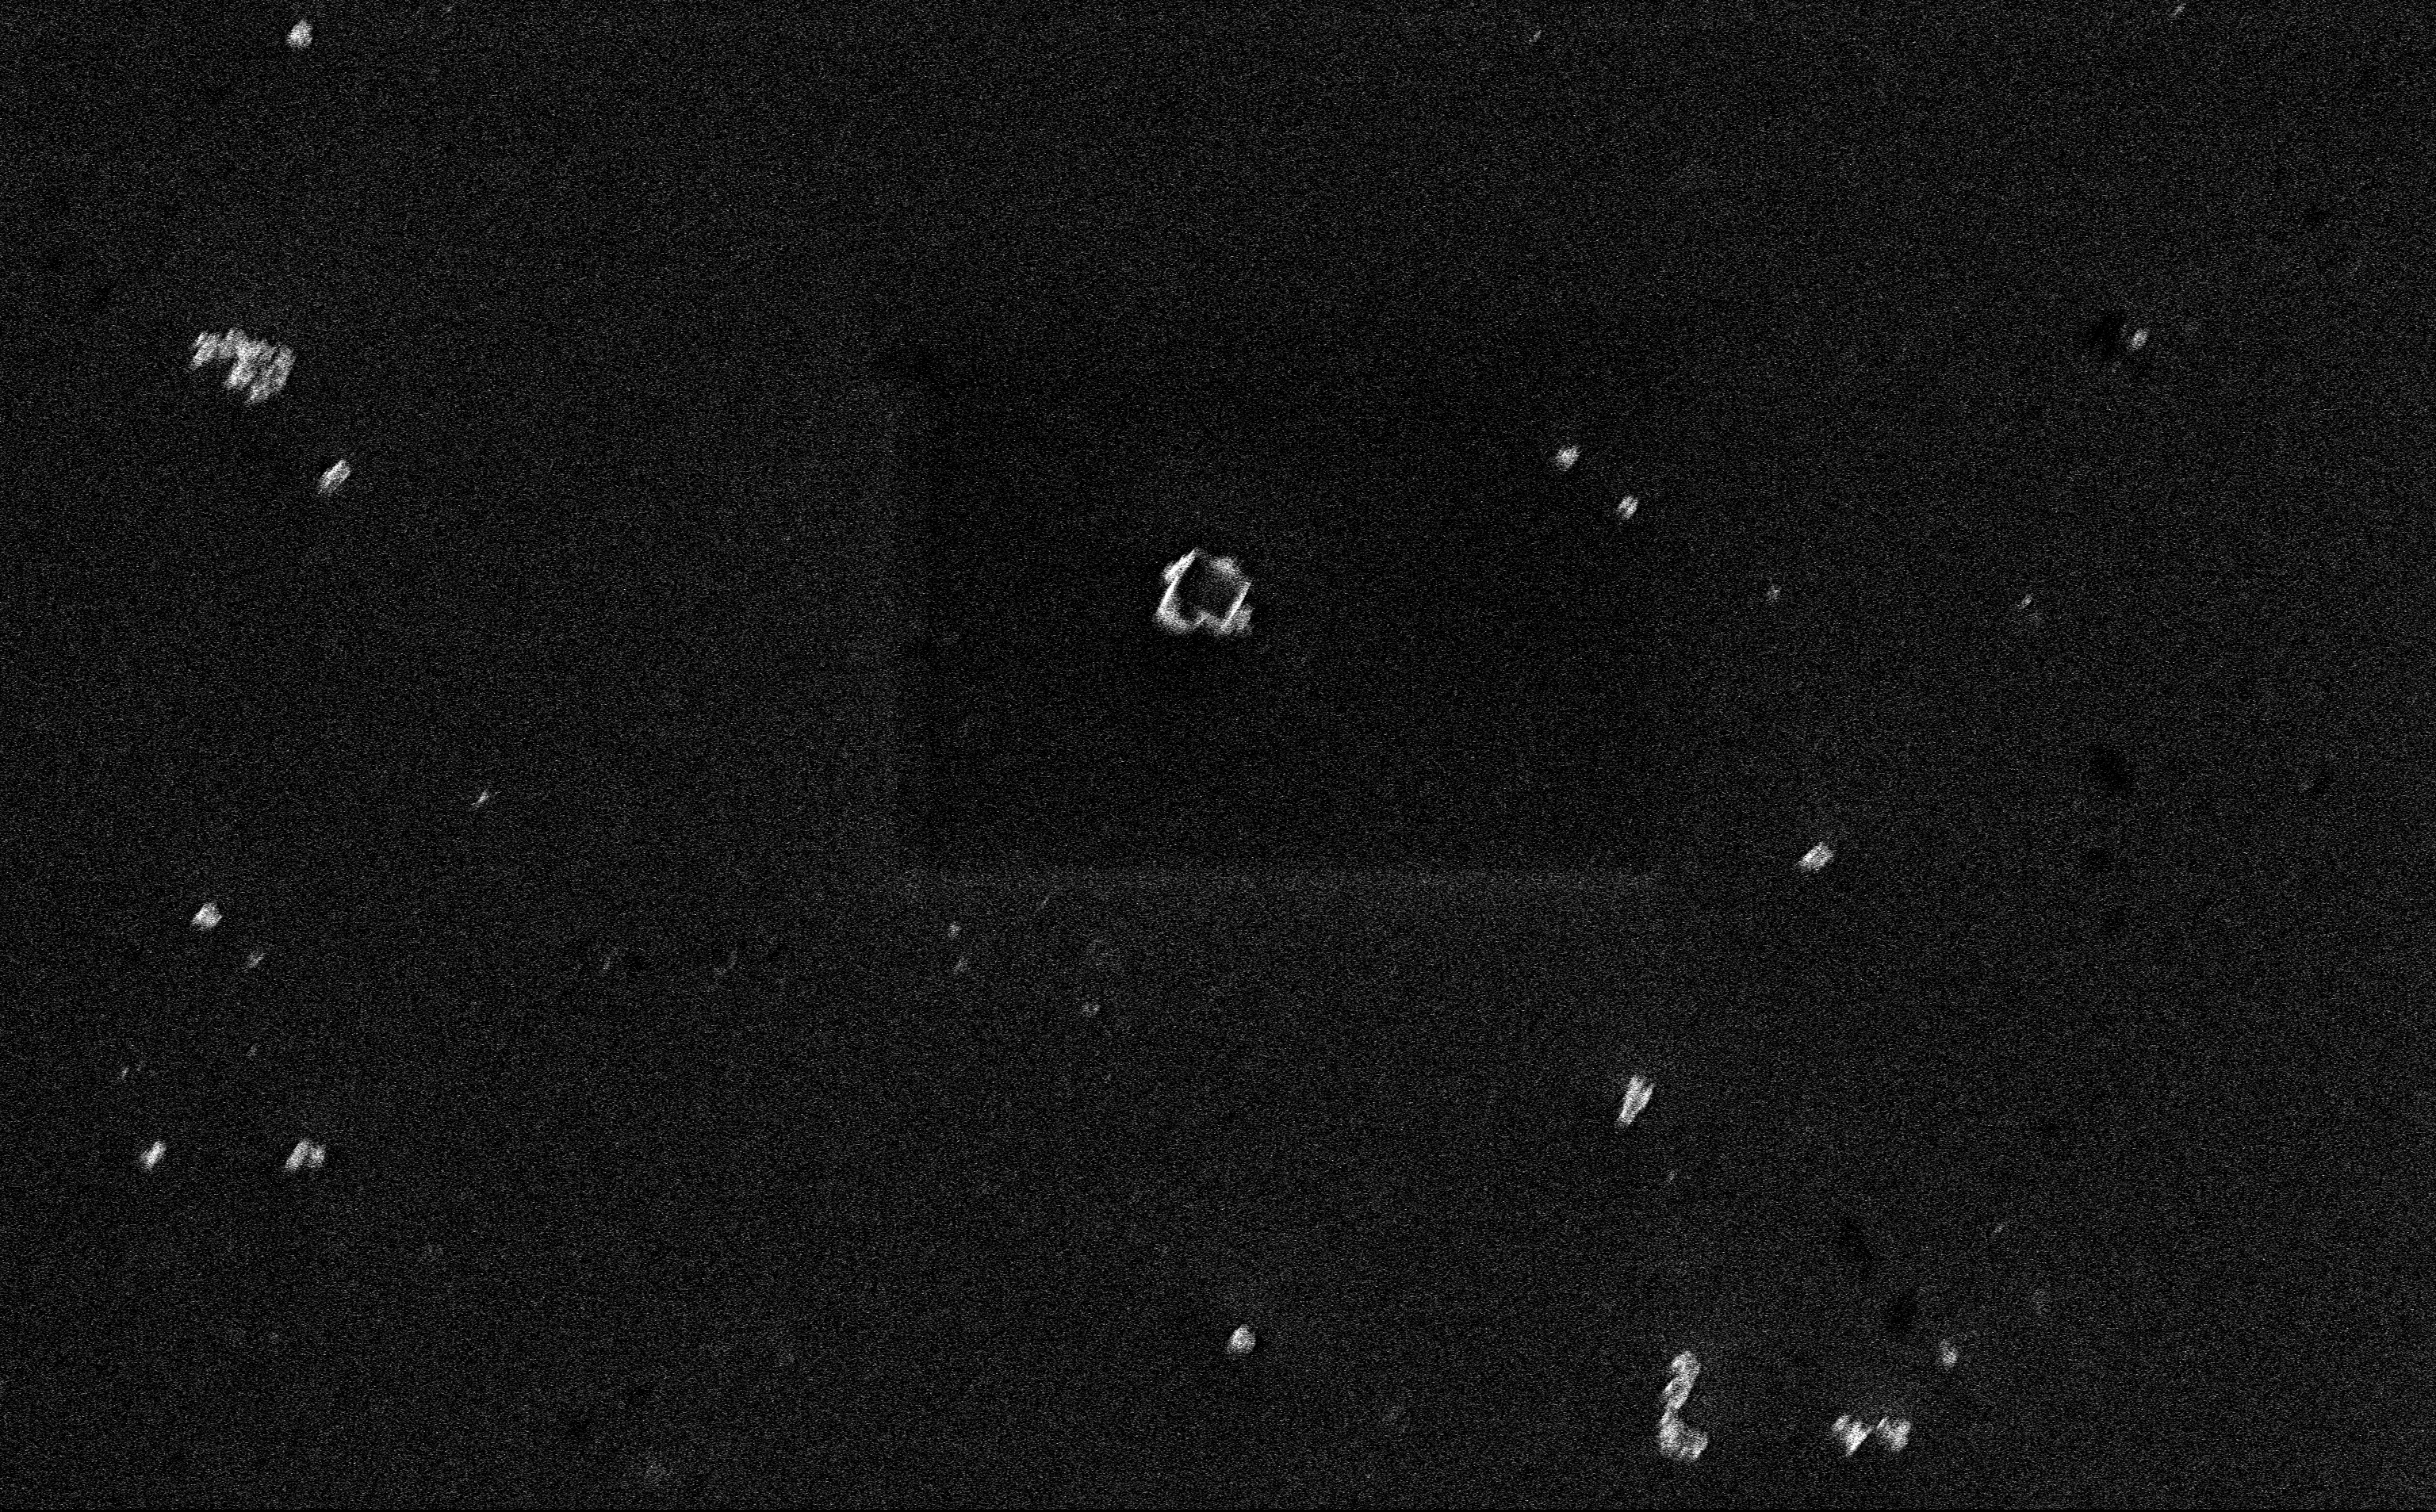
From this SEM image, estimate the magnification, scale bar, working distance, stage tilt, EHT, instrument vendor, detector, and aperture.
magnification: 12.85 K X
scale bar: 2000 nm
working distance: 3 mm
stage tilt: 0°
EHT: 3 kV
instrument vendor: Zeiss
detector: InLens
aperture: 30 µm